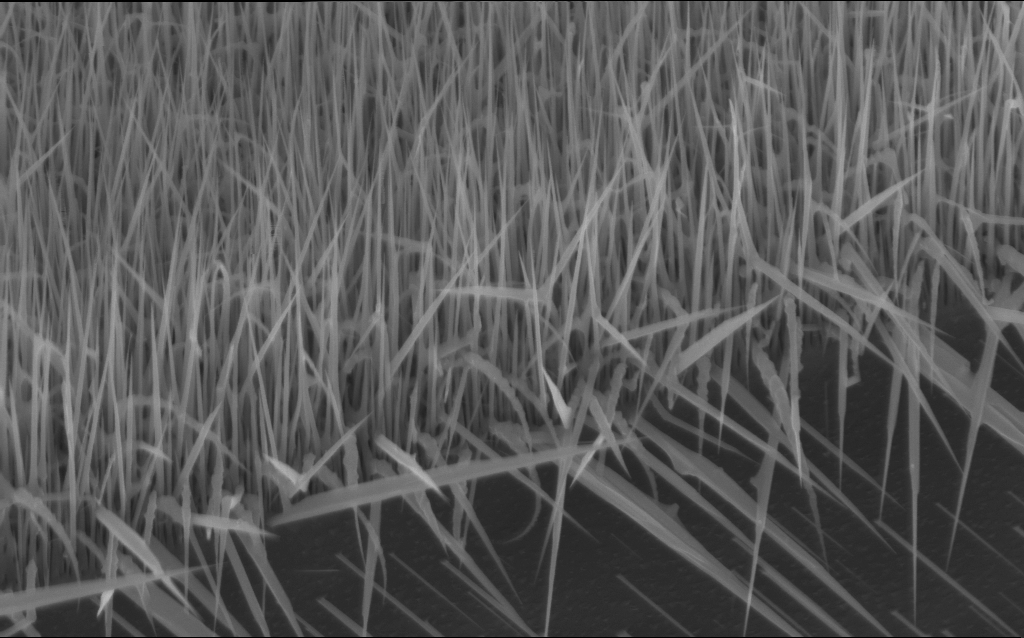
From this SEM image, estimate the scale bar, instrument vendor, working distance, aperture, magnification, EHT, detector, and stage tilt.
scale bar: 1000 nm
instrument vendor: Zeiss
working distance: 6 mm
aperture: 30 µm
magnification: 40 K X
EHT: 10 kV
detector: InLens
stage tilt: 45°